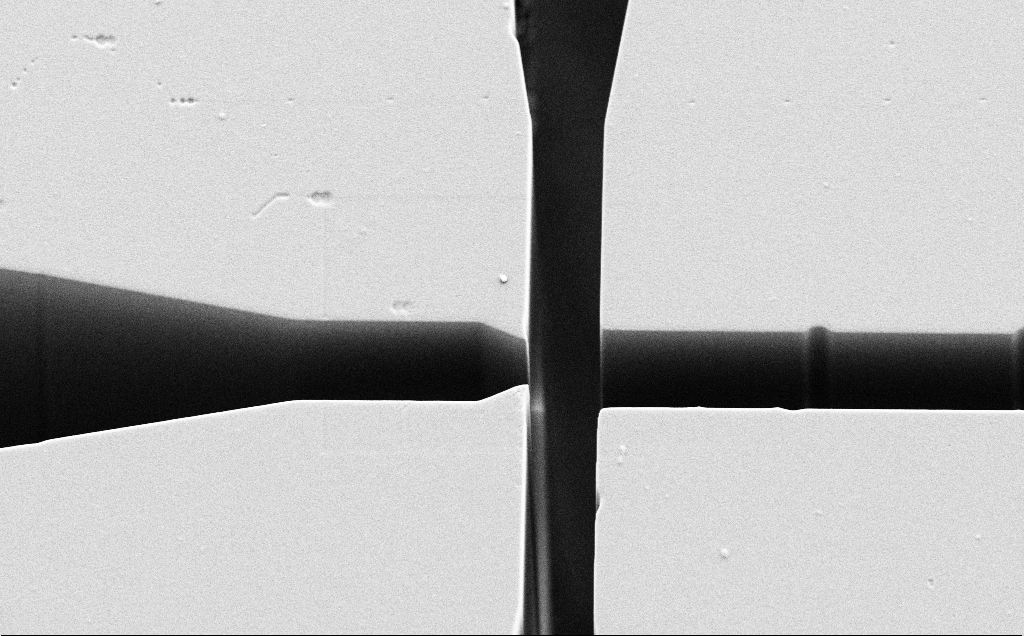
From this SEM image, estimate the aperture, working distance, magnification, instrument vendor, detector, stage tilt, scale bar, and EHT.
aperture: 30 µm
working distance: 7 mm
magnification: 2.28 K X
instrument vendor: Zeiss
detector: SE2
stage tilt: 25°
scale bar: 20000 nm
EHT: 5 kV